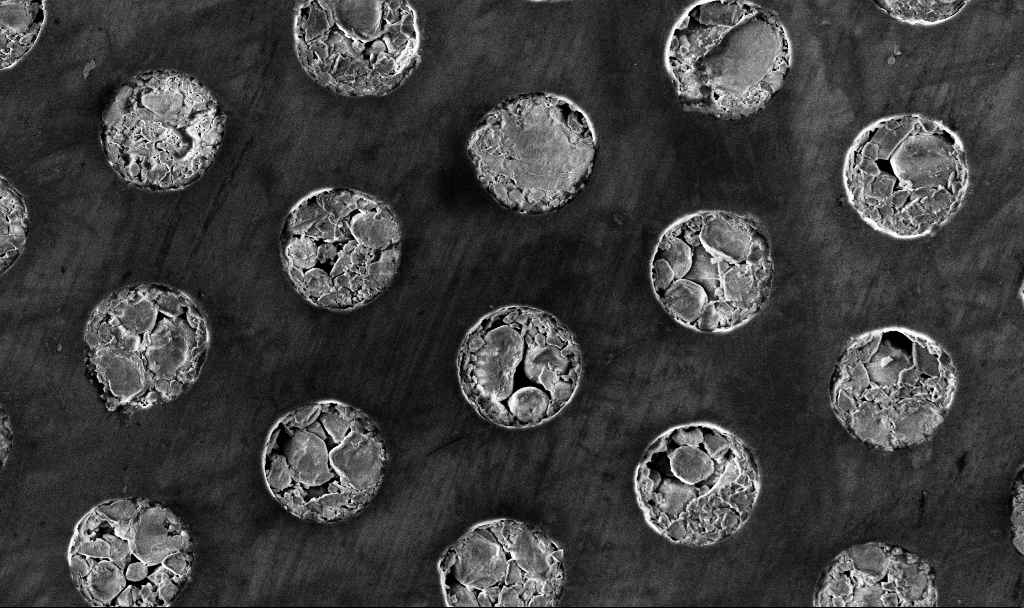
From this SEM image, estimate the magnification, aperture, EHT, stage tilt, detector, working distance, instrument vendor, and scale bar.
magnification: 13.19 K X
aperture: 30 µm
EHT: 3 kV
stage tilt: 0°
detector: InLens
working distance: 4.9 mm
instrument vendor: Zeiss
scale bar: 2000 nm